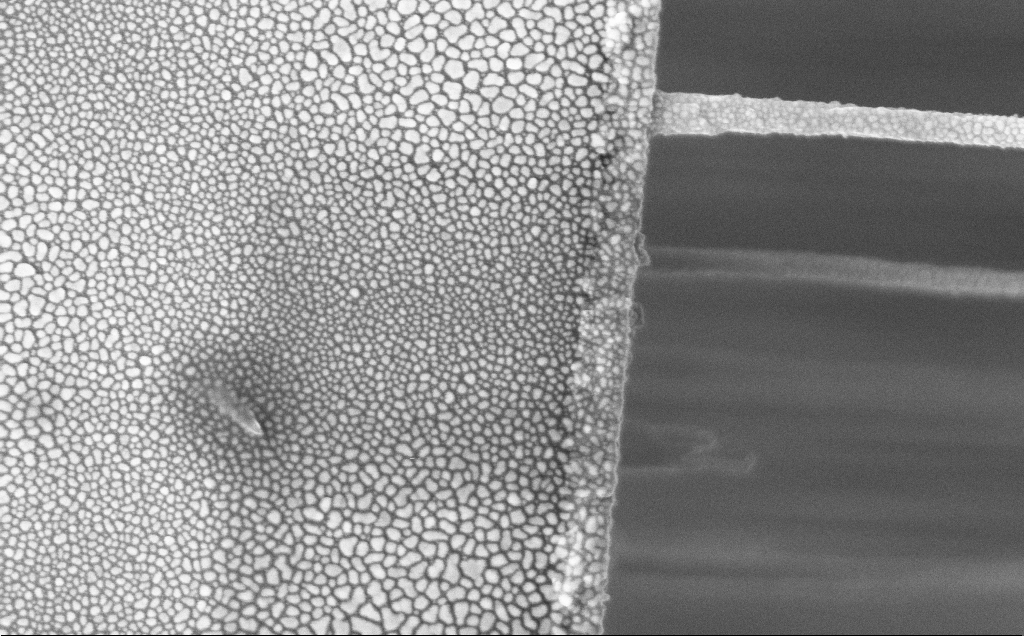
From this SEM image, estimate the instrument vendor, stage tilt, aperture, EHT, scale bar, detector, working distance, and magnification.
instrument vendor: Zeiss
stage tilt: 0°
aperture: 30 µm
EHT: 5 kV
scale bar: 200 nm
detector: InLens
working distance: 12 mm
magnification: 77.16 K X